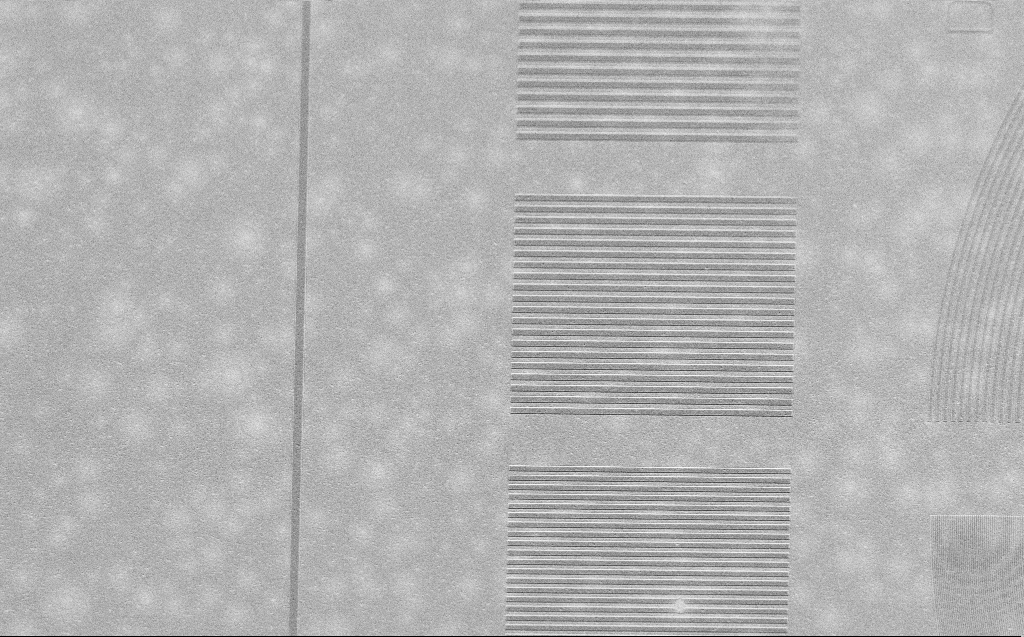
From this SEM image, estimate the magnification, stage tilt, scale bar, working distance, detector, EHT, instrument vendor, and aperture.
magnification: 3.5 K X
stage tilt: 30°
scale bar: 10000 nm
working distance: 4 mm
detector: SE2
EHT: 2.5 kV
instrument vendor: Zeiss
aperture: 30 µm